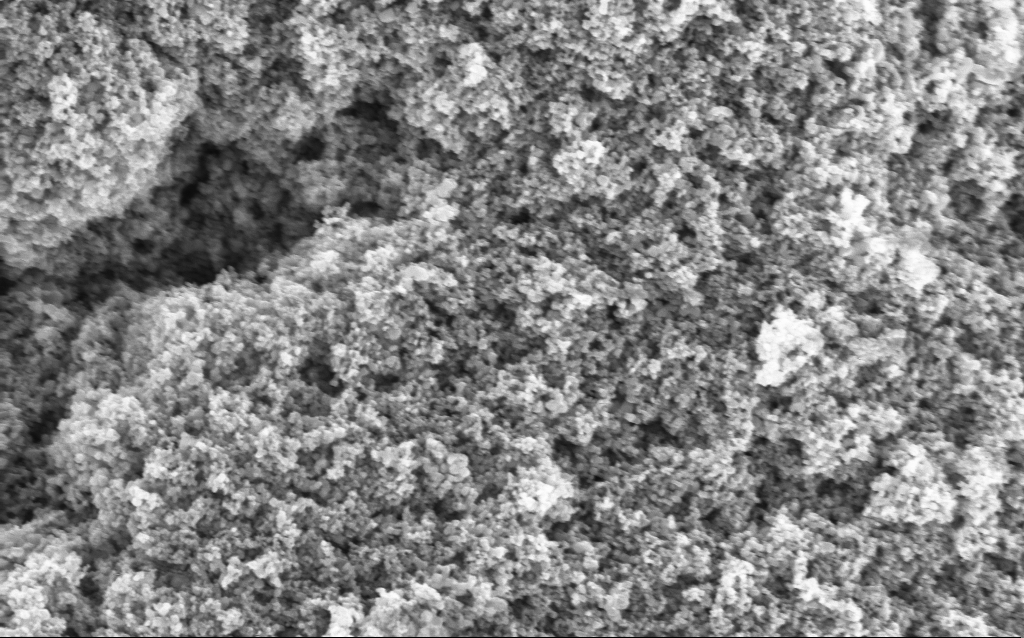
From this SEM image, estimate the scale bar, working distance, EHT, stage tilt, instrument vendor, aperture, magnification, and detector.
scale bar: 1000 nm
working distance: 4.5 mm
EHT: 5 kV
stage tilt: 0°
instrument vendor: Zeiss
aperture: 30 µm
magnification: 68.64 K X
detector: InLens